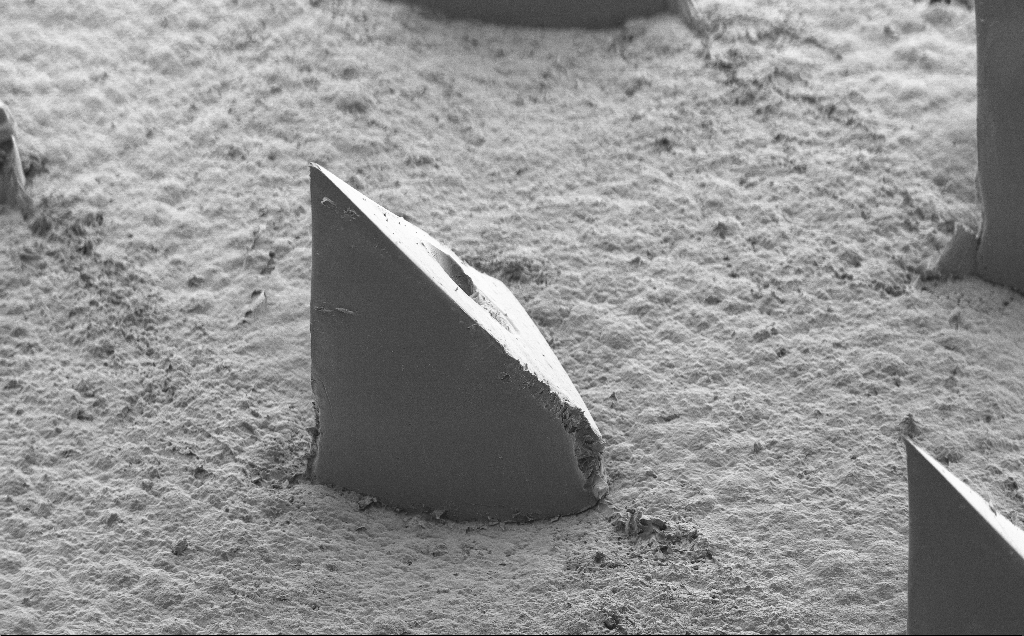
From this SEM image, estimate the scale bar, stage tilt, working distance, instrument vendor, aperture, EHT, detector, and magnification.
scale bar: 100000 nm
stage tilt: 40°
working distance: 9 mm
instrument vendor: Zeiss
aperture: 30 µm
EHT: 5 kV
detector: SE2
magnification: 0.183 K X